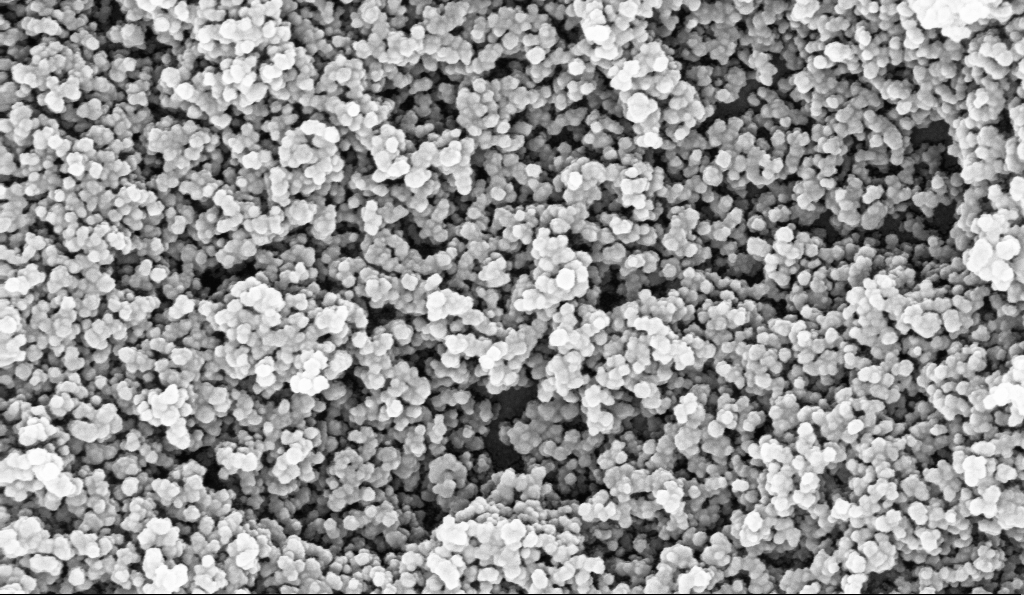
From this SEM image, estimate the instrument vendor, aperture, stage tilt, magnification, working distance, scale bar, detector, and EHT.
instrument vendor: Zeiss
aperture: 30 µm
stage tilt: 0°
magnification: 135 K X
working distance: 5.1 mm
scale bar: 200 nm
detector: InLens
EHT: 10 kV